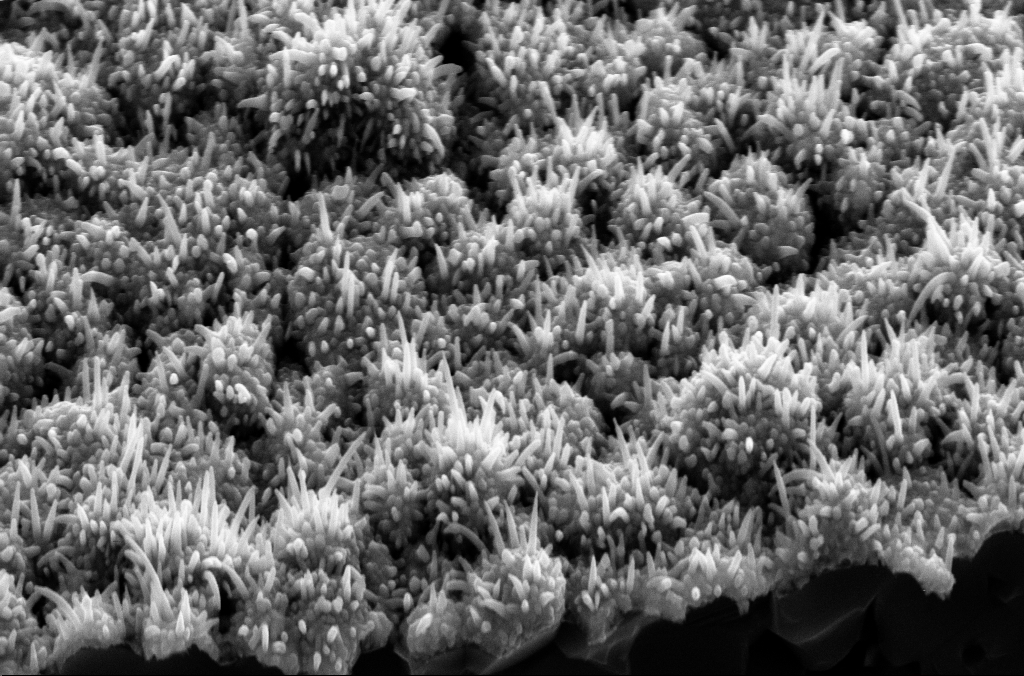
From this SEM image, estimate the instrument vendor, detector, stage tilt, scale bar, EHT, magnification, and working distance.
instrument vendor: Zeiss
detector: SE2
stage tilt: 45°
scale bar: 1000 nm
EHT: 10 kV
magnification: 41.39 K X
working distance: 8 mm